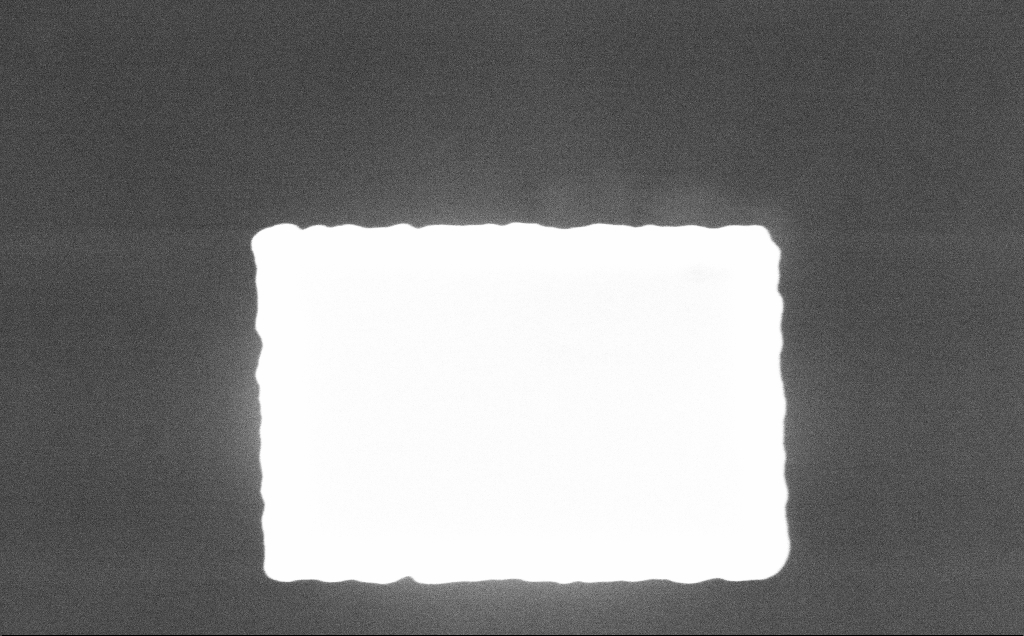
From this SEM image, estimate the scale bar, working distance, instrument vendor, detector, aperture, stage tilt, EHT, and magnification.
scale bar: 1000 nm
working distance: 4 mm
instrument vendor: Zeiss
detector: InLens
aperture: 30 µm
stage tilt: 0°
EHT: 3 kV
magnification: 62.68 K X